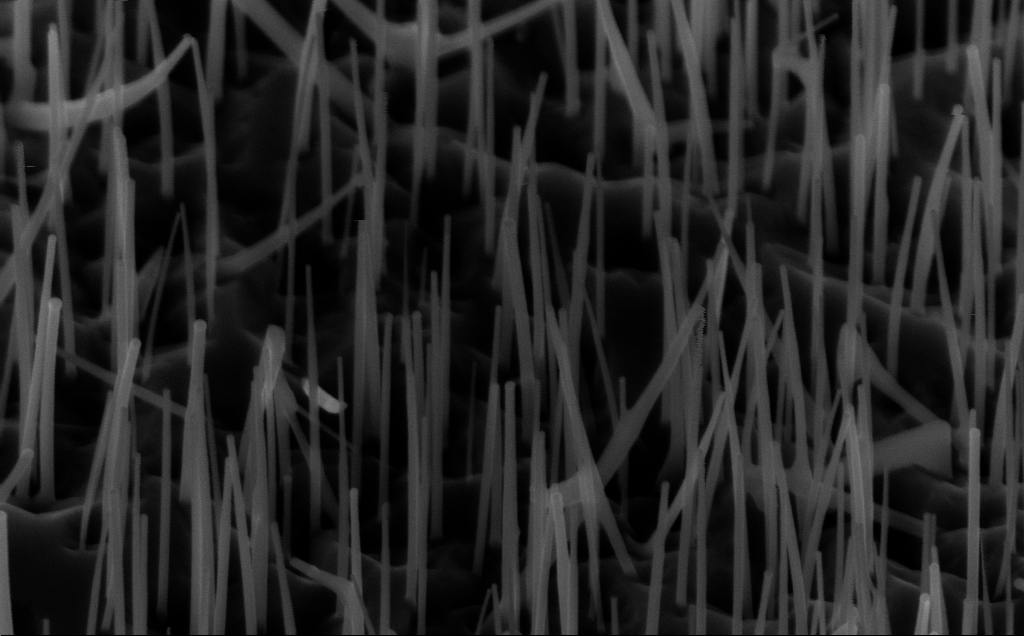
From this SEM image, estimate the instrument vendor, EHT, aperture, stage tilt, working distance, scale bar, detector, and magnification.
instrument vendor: Zeiss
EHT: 10 kV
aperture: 30 µm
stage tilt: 45°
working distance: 5 mm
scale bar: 200 nm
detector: InLens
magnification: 80 K X